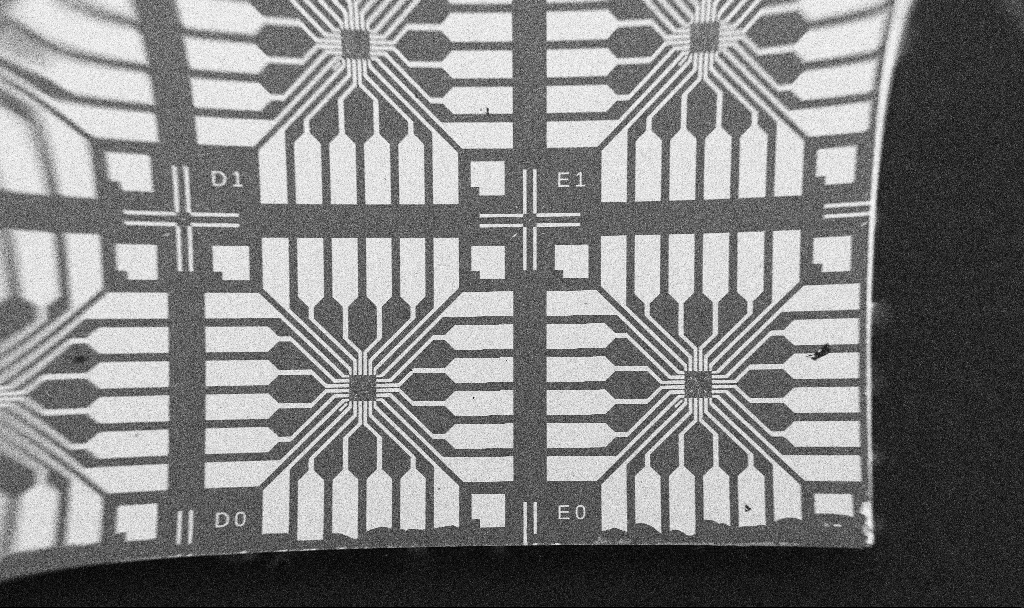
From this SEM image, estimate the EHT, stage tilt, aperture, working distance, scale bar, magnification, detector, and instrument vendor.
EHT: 5 kV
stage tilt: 0°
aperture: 30 µm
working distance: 10.7 mm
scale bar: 1e+06 nm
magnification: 0.061 K X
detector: SE2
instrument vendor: Zeiss